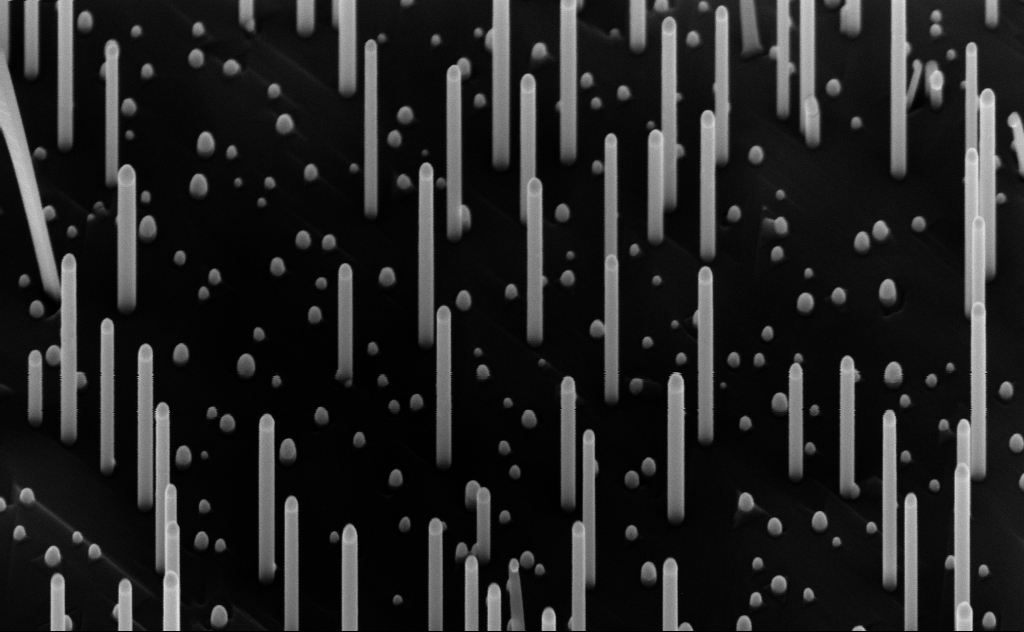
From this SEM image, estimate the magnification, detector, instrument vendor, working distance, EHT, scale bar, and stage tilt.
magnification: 80 K X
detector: InLens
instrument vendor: Zeiss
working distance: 7 mm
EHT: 10 kV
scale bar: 200 nm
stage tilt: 45°